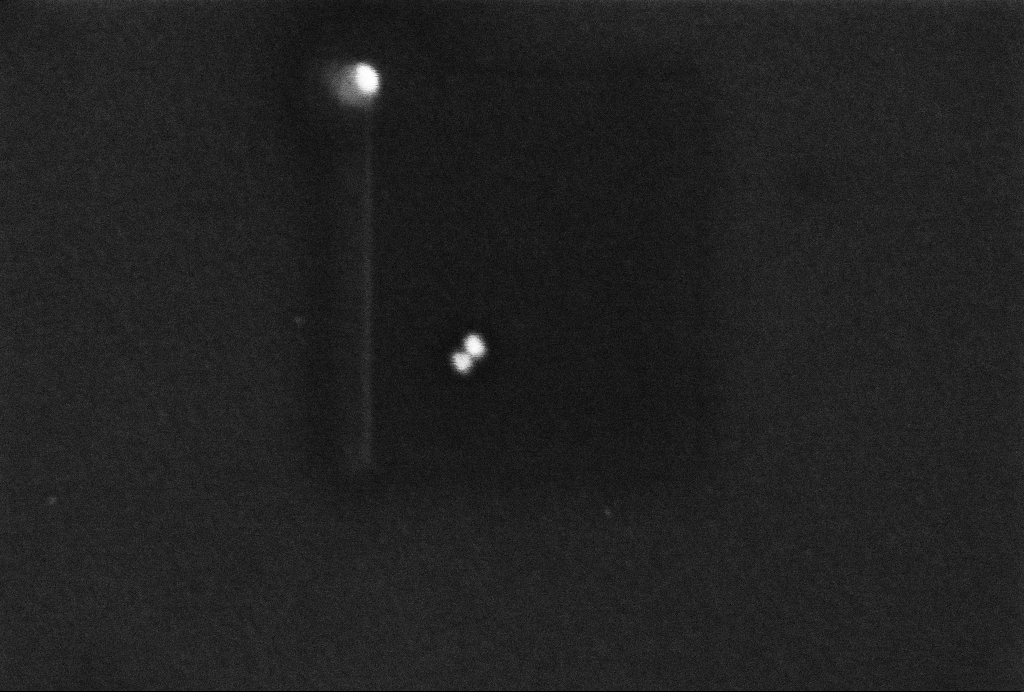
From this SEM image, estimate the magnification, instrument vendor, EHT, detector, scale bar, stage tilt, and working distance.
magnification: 276.16 K X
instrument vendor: Zeiss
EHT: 2 kV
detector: InLens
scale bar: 200 nm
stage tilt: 0°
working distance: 3.3 mm